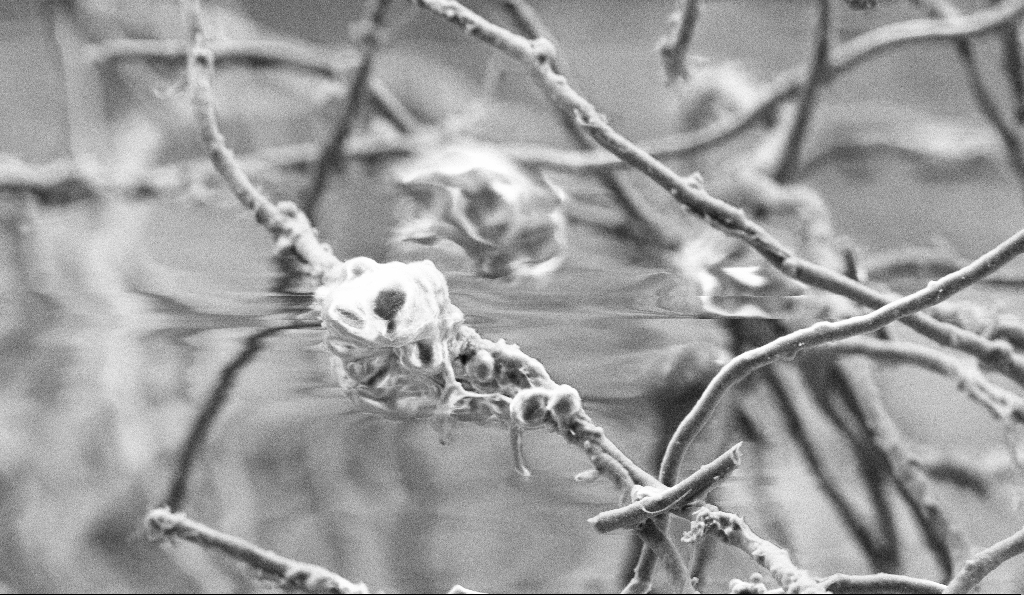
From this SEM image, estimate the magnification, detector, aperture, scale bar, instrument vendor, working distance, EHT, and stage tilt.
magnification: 10 K X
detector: SE2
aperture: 30 µm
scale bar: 2000 nm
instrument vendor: Zeiss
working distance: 4.9 mm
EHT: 3 kV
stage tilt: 0°